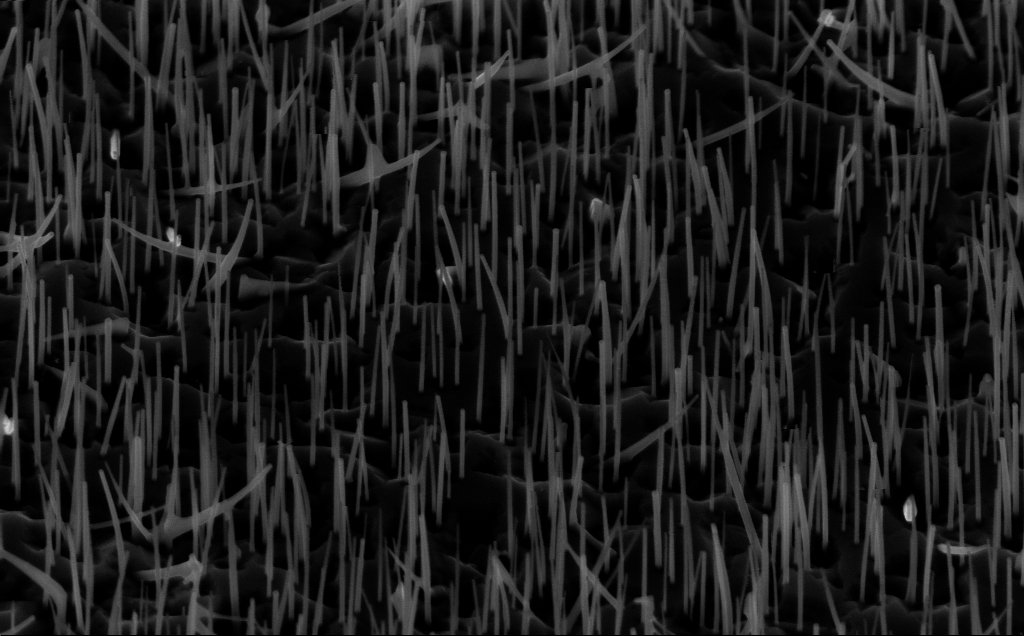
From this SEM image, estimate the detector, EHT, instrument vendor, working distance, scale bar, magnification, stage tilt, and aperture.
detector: InLens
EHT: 10 kV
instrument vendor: Zeiss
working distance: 5 mm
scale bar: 1000 nm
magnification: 40 K X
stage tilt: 45°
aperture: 30 µm